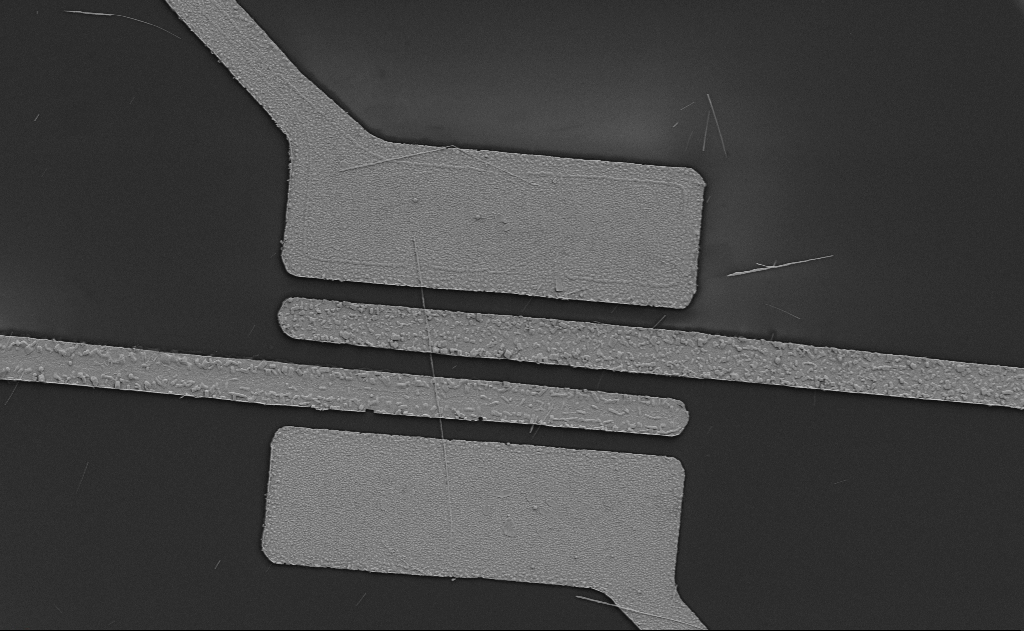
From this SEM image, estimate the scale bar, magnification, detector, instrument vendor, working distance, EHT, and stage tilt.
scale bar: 10000 nm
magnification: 5 K X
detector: SE2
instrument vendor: Zeiss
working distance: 13 mm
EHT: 5 kV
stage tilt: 0°